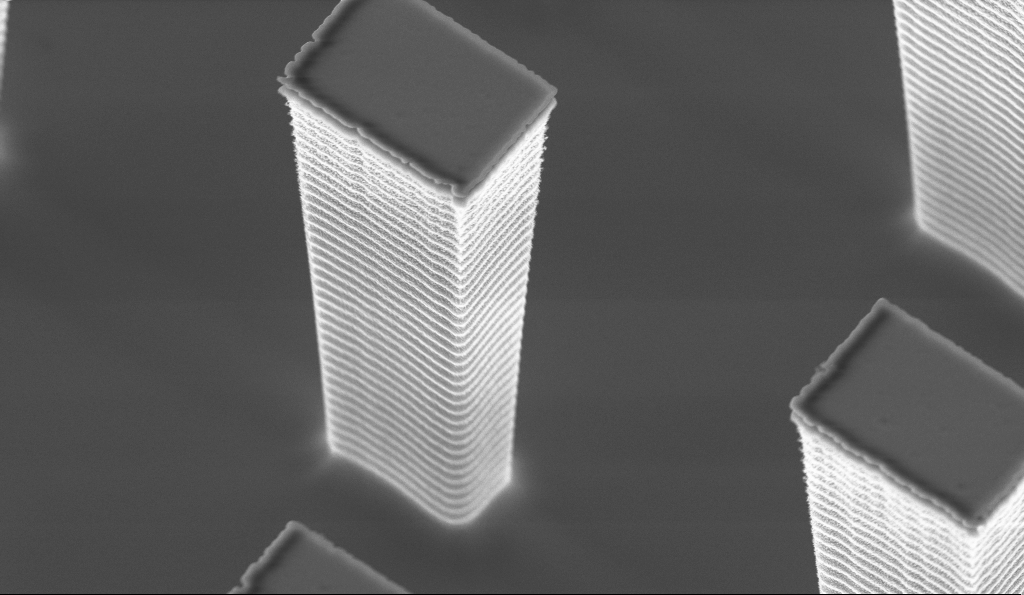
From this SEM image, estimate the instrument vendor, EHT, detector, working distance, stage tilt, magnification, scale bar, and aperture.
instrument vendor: Zeiss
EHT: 5 kV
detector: InLens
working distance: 5.3 mm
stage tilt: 30°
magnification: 19.48 K X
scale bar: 2000 nm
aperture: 30 µm